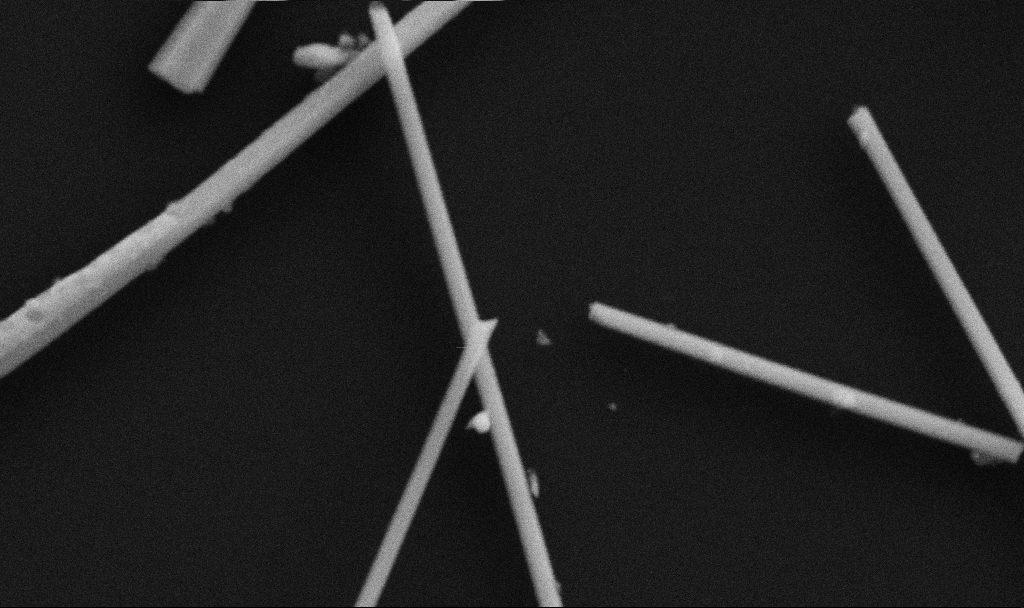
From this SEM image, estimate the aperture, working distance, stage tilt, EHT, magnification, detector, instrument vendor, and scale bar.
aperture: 30 µm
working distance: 6.7 mm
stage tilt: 0°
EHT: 5 kV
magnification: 100.91 K X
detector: SE2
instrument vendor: Zeiss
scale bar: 200 nm